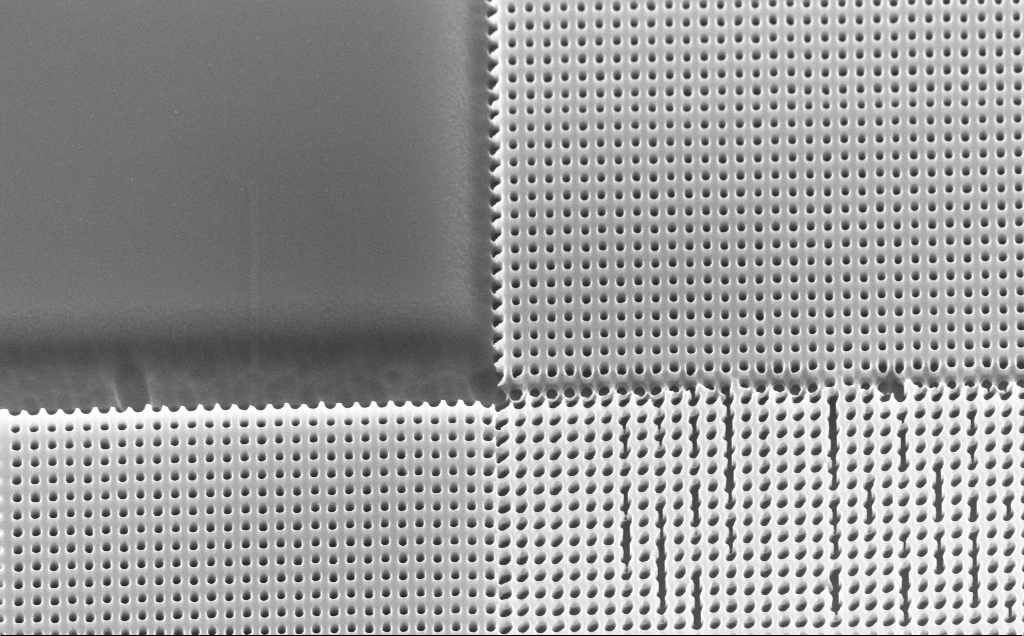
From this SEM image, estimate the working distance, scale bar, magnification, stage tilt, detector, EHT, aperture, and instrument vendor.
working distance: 4 mm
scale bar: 1000 nm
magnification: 42.72 K X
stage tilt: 30°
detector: InLens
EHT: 10 kV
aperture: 30 µm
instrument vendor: Zeiss